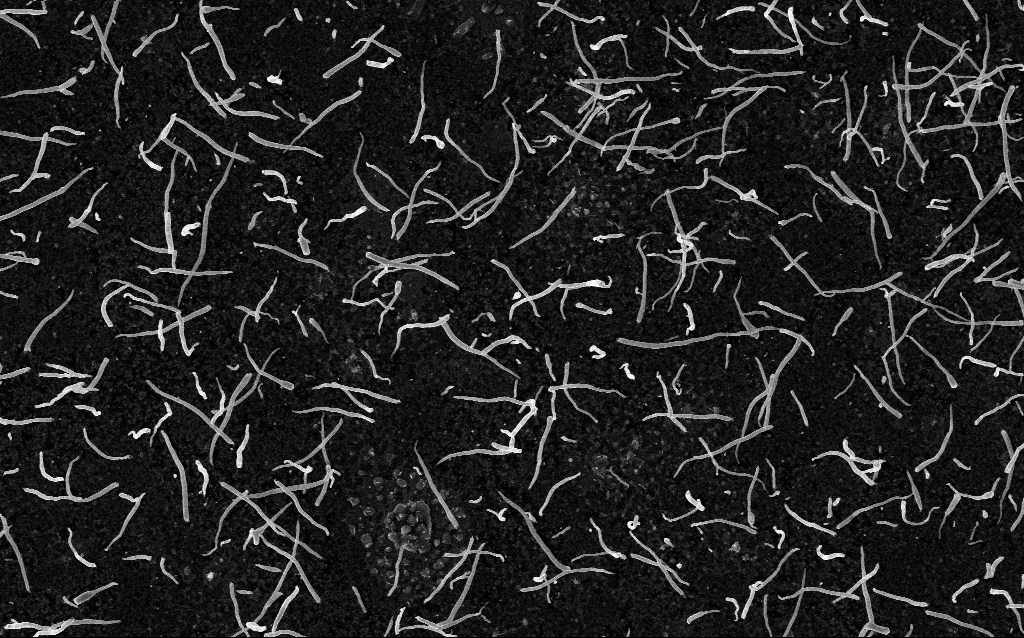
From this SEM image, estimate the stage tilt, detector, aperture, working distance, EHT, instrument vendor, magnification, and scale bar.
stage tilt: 0°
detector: InLens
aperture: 30 µm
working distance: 2 mm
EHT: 5 kV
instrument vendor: Zeiss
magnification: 20 K X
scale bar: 1000 nm